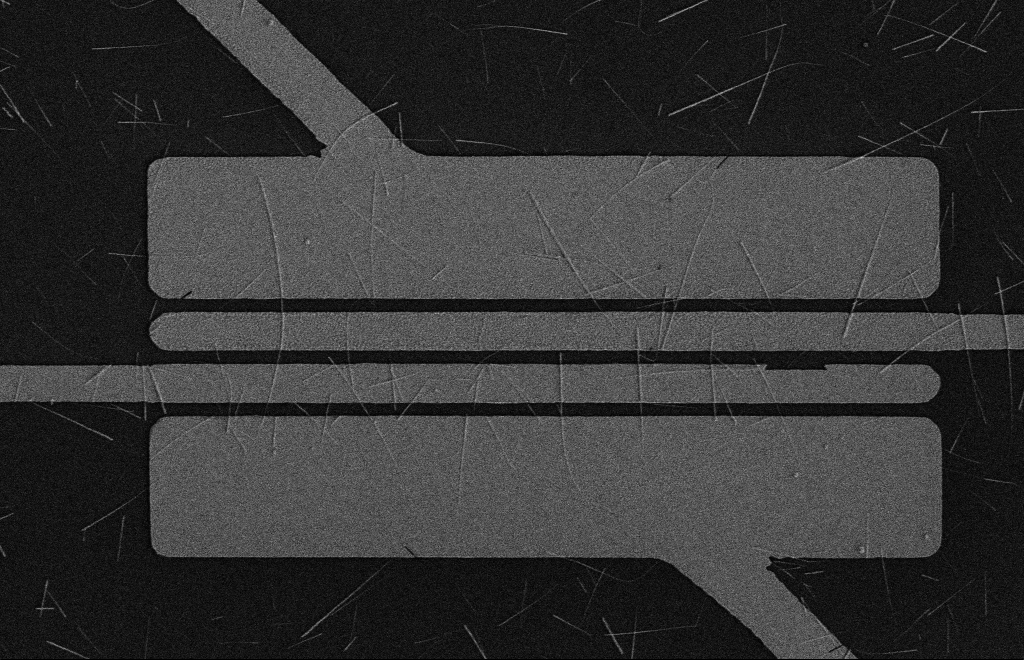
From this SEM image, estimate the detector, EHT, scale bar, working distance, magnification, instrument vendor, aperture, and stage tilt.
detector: SE2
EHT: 5 kV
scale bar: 2000 nm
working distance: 16 mm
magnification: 4.79 K X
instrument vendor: Zeiss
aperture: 10 µm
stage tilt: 0°